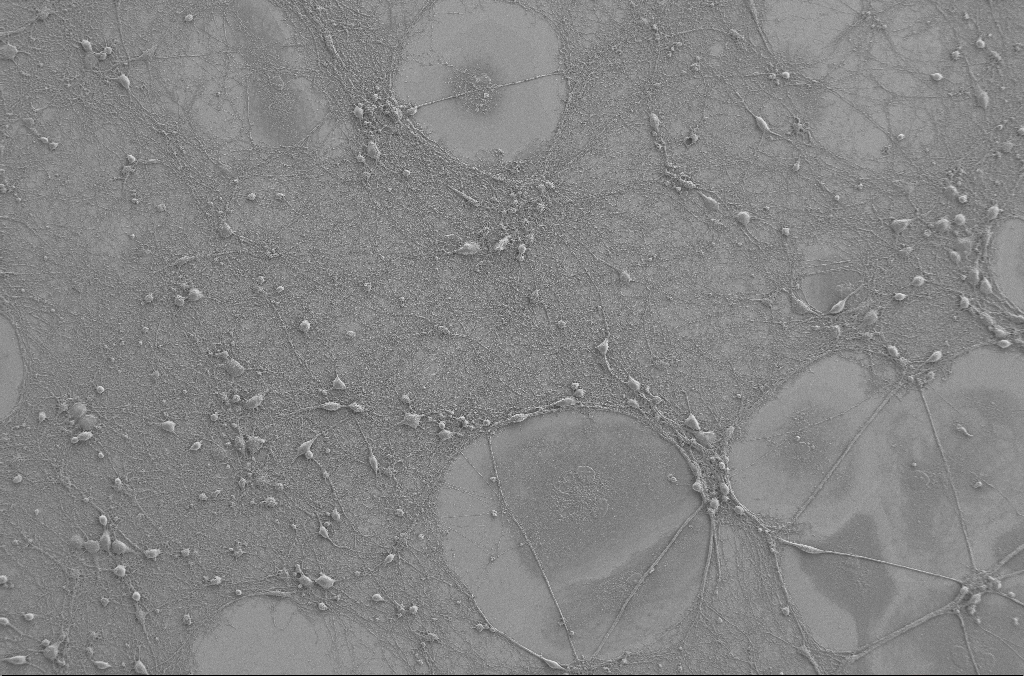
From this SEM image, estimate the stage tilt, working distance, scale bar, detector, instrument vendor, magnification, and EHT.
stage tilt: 0°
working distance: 4 mm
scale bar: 100000 nm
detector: SE2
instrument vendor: Zeiss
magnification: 0.5 K X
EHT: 2 kV